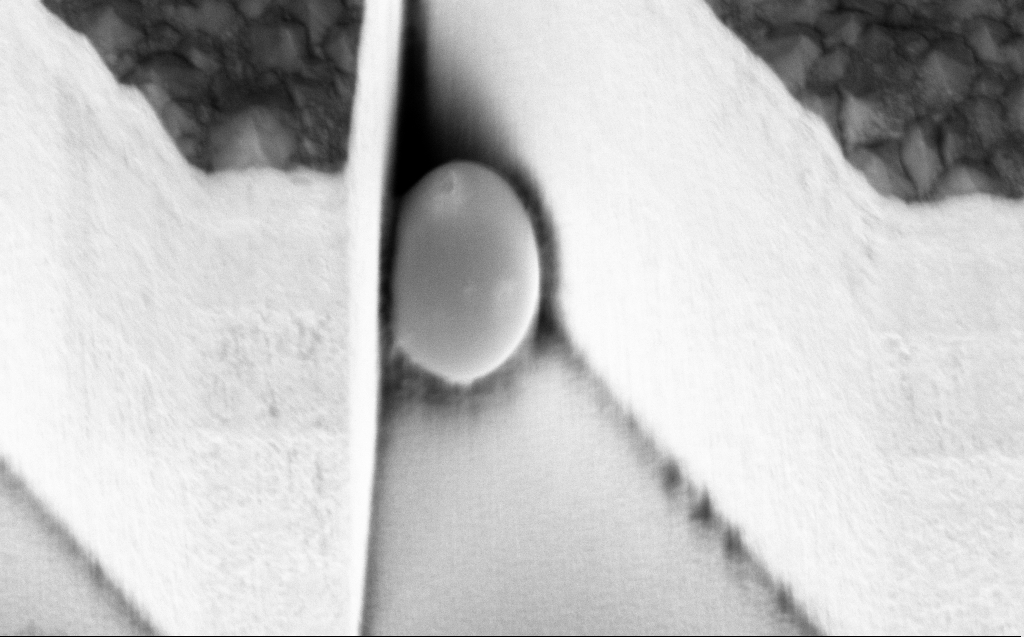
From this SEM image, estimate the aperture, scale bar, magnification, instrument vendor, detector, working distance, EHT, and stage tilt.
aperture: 120 µm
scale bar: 2000 nm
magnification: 21.84 K X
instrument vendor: Zeiss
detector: InLens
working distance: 7 mm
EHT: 3 kV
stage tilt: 45.1°